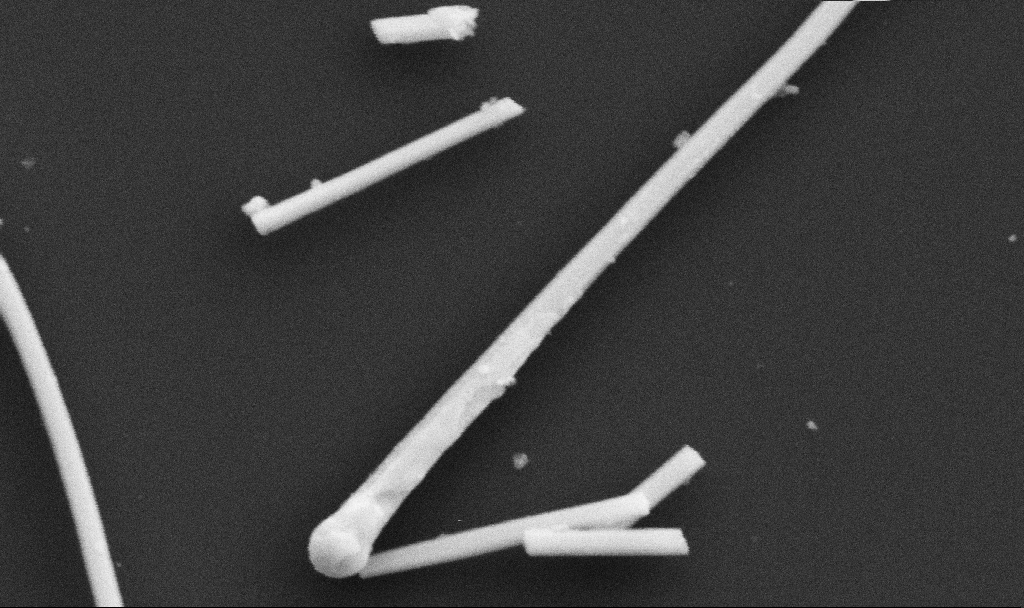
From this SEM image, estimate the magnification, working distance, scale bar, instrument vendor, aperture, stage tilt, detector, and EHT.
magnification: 100.56 K X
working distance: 10.7 mm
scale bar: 200 nm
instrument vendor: Zeiss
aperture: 30 µm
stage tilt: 0°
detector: SE2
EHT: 5 kV